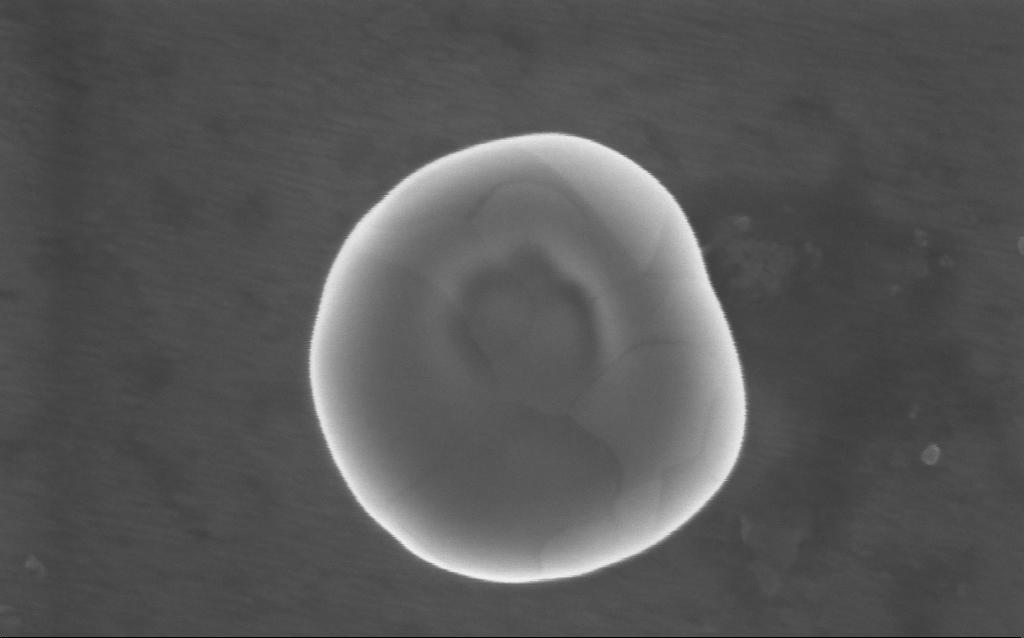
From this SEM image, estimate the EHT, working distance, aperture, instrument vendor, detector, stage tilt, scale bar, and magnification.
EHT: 5 kV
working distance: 4 mm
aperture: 30 µm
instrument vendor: Zeiss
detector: InLens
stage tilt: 0°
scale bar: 200 nm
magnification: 232 K X